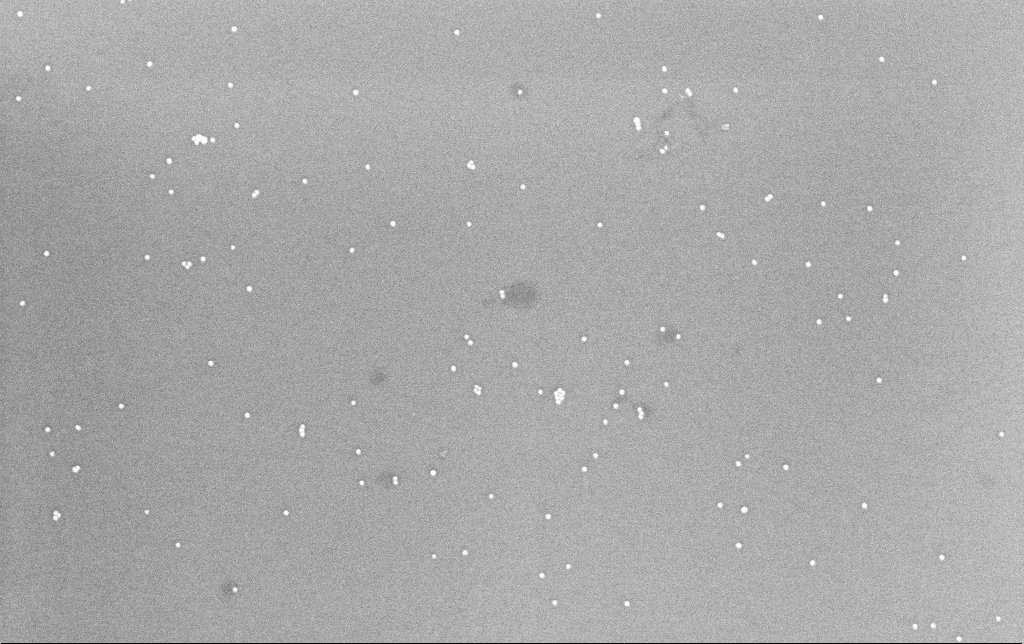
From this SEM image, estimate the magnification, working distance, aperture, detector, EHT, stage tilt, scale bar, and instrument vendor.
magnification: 100 K X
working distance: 6.6 mm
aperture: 30 µm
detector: InLens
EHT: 8 kV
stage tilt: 0°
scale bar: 200 nm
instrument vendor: Zeiss